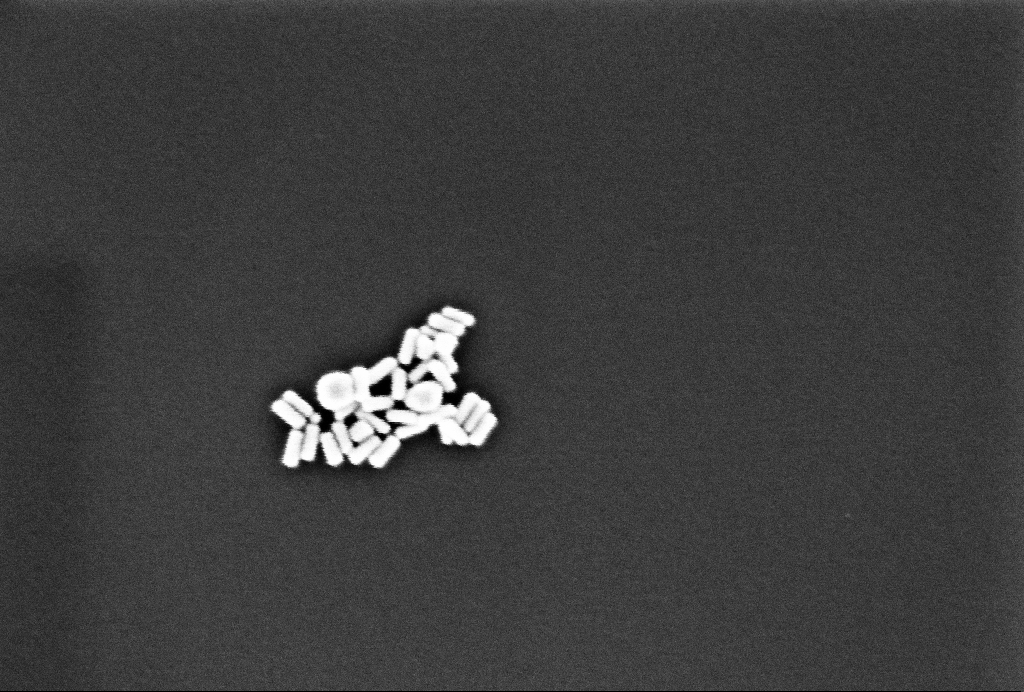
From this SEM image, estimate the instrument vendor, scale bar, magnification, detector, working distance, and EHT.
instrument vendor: Zeiss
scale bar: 200 nm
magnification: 192.65 K X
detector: InLens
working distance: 3.3 mm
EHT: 2 kV